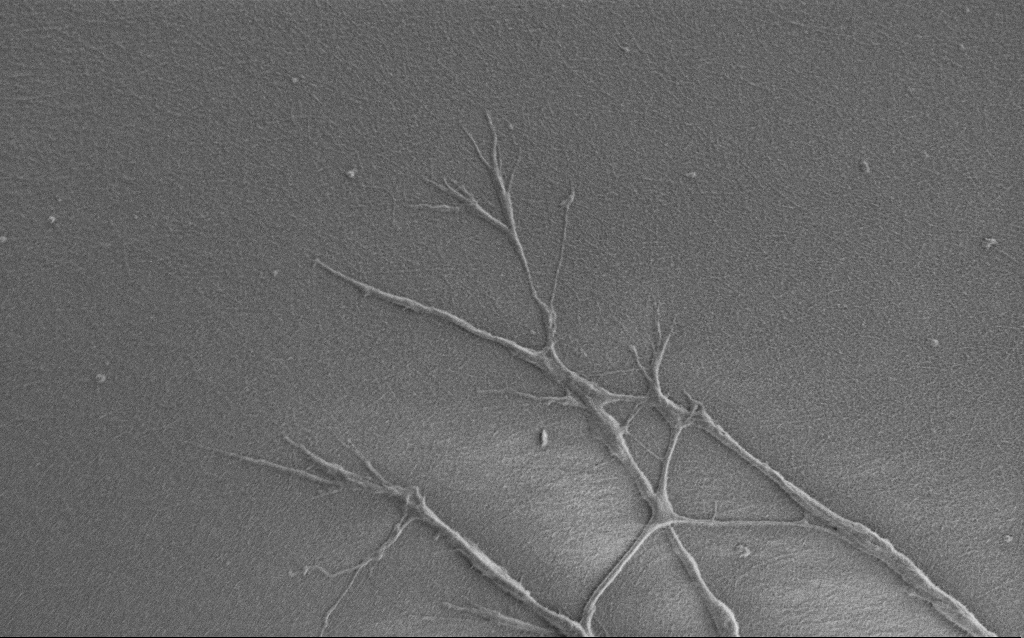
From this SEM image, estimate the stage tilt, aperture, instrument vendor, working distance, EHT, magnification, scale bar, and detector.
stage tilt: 0°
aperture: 30 µm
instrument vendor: Zeiss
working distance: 7 mm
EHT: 0.9 kV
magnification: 6 K X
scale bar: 10000 nm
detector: SE2